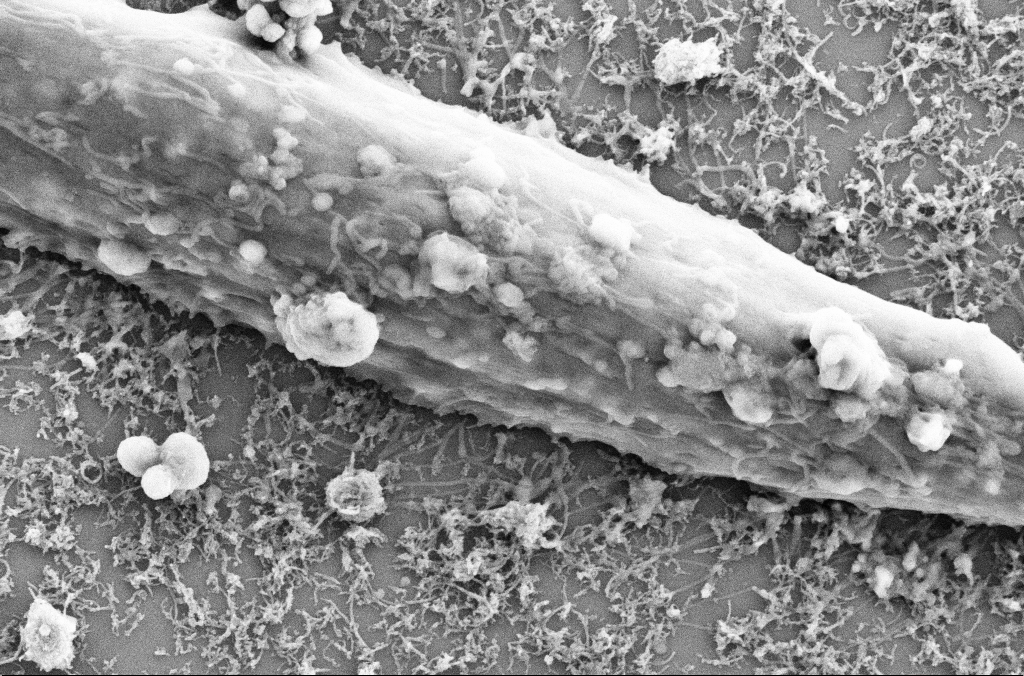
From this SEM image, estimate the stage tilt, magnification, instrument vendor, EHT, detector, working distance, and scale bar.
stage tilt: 0°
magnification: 20 K X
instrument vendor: Zeiss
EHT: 5 kV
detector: SE2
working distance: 4 mm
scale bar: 1000 nm